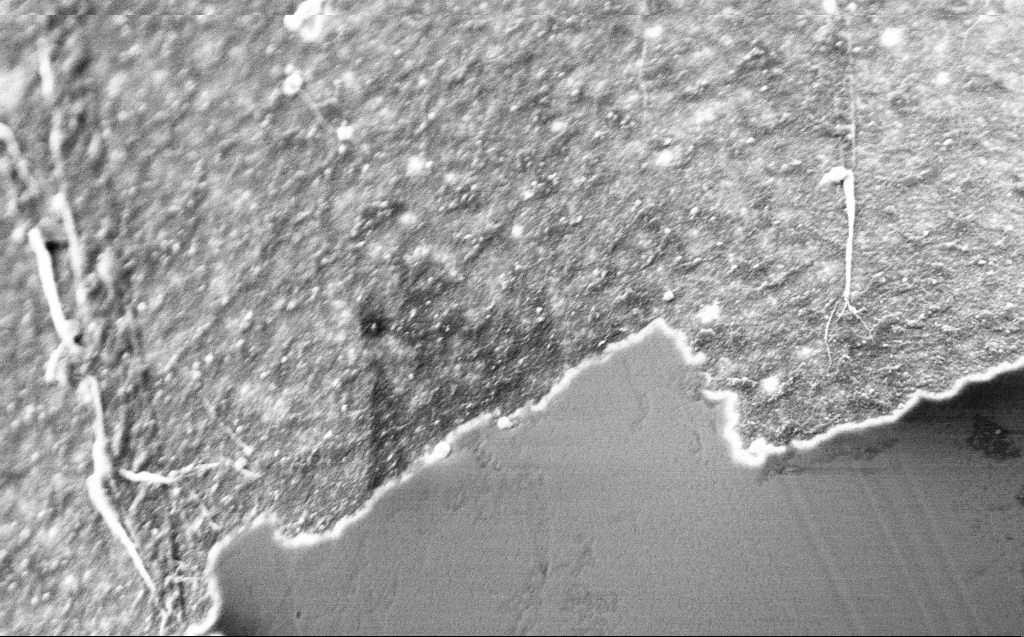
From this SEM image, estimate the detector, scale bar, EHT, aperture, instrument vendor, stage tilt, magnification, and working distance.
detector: InLens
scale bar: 1000 nm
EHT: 2 kV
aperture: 30 µm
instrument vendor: Zeiss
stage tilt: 45°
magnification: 50 K X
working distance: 3 mm